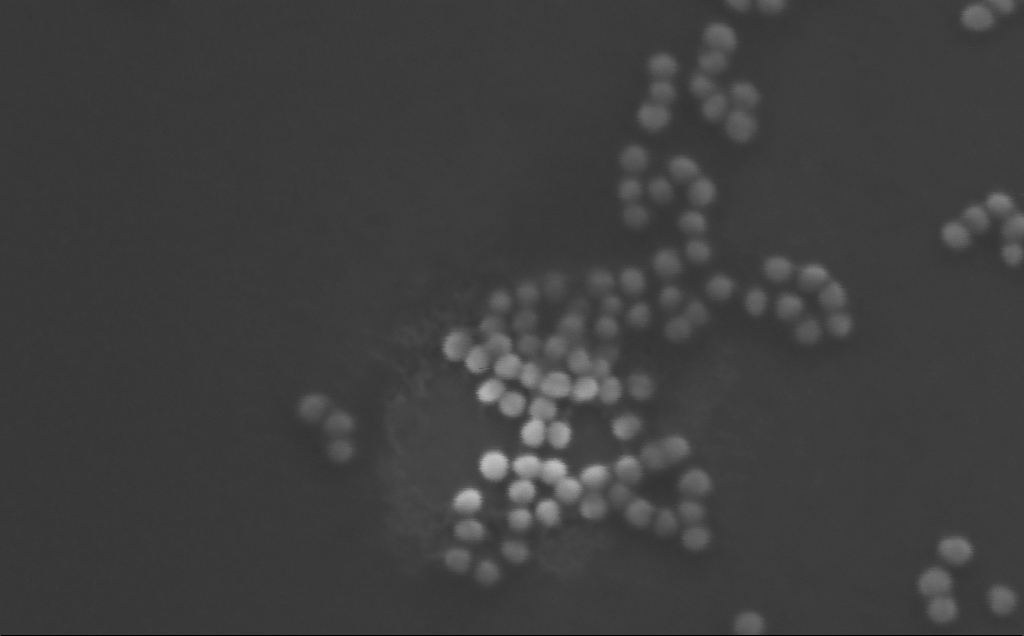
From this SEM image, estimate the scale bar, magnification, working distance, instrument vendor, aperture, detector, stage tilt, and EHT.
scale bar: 100 nm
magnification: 631.89 K X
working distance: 4 mm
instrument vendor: Zeiss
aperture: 30 µm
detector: InLens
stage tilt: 25°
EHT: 10 kV